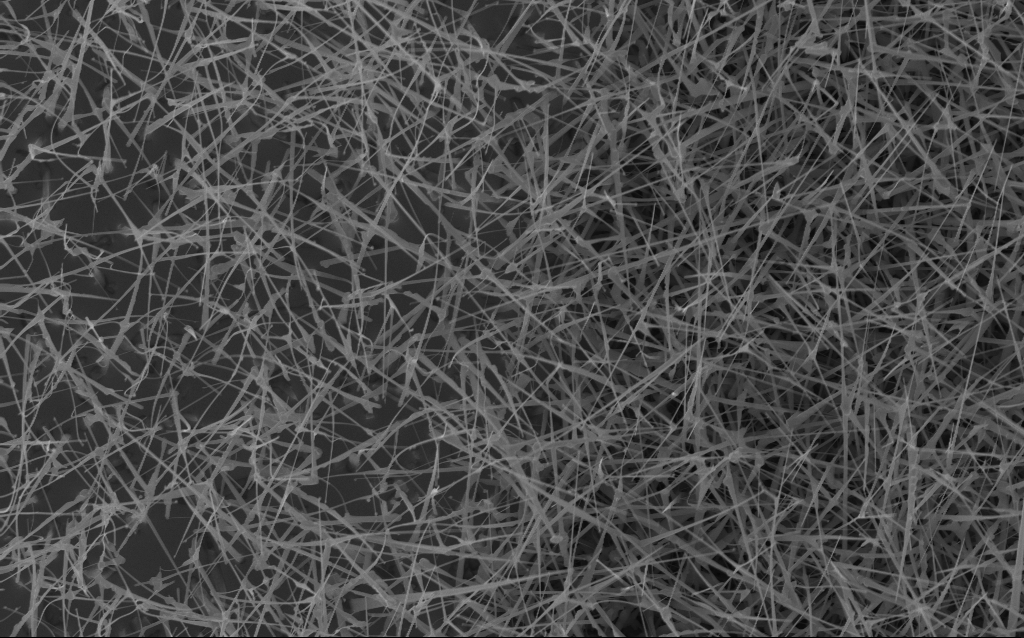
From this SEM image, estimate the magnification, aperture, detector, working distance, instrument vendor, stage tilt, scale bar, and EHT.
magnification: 10 K X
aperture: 30 µm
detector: InLens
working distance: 6 mm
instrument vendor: Zeiss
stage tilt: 0°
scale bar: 2000 nm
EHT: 10 kV